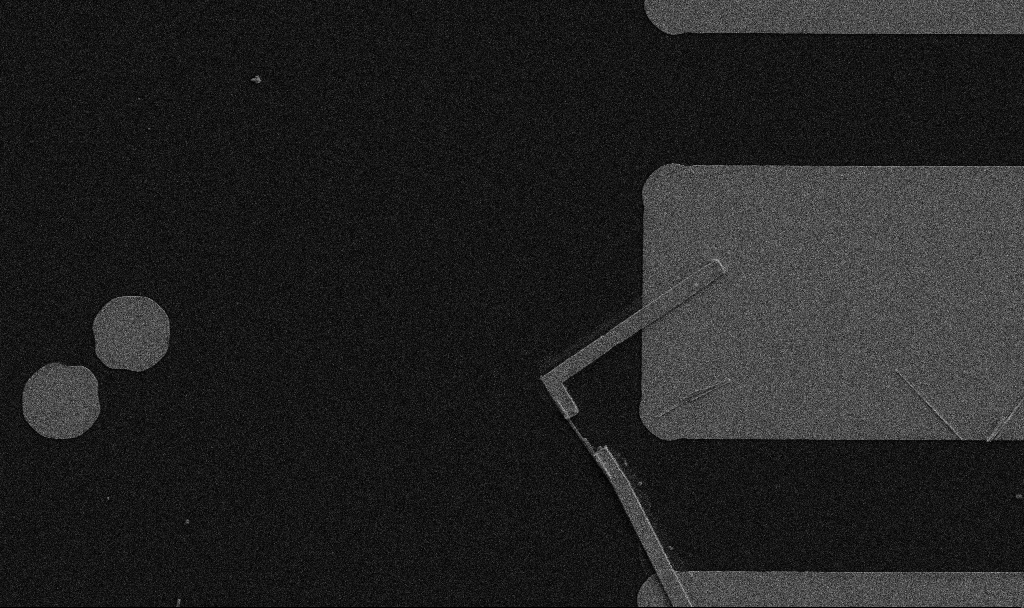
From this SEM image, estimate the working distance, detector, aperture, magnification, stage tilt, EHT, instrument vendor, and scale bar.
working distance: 10.7 mm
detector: SE2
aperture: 30 µm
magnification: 5 K X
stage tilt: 0°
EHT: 5 kV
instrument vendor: Zeiss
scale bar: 10000 nm